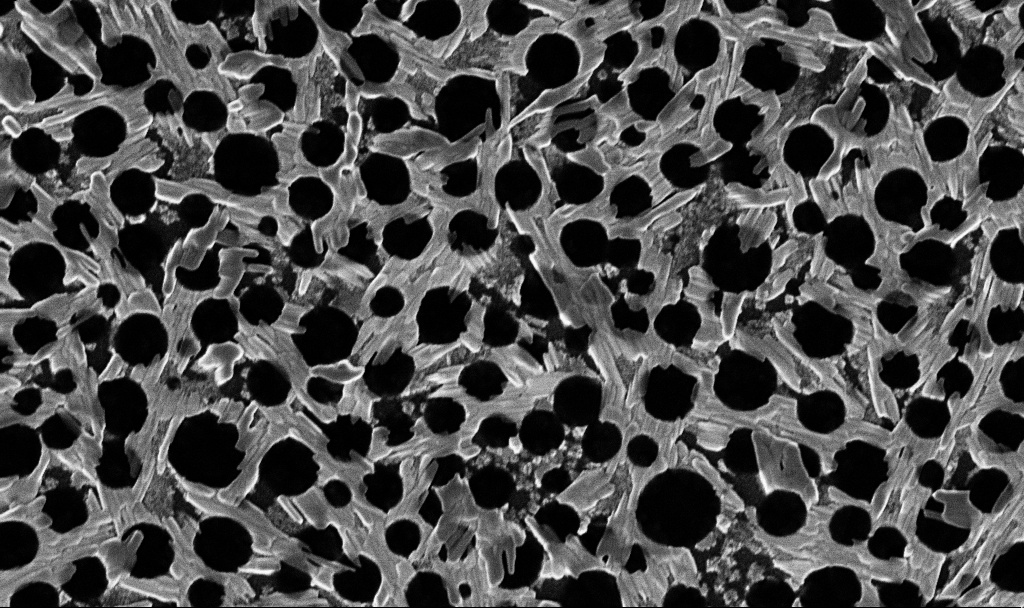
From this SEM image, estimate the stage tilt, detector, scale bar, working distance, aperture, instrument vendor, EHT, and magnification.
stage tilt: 0°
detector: InLens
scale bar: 2000 nm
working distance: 3.3 mm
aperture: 30 µm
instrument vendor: Zeiss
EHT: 2 kV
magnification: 20 K X